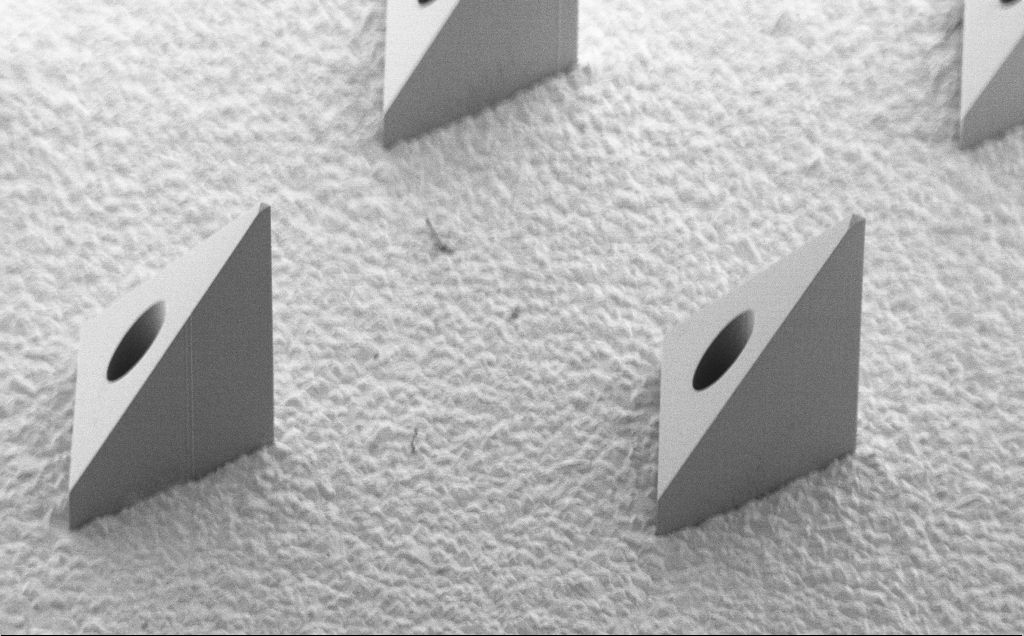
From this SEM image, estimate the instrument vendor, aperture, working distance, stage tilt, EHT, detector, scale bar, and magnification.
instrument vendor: Zeiss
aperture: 30 µm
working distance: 8 mm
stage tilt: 40°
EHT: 5 kV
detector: SE2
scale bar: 200000 nm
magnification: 0.139 K X